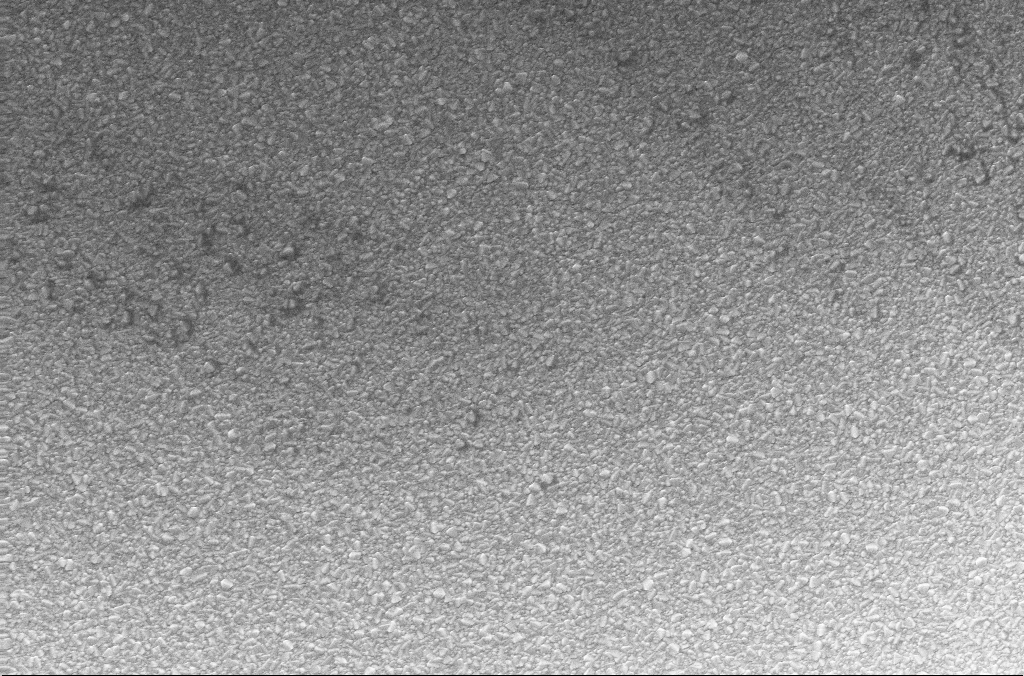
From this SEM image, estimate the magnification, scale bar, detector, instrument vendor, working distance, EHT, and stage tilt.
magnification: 10 K X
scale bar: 2000 nm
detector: InLens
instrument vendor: Zeiss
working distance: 1.9 mm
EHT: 20 kV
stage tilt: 0°